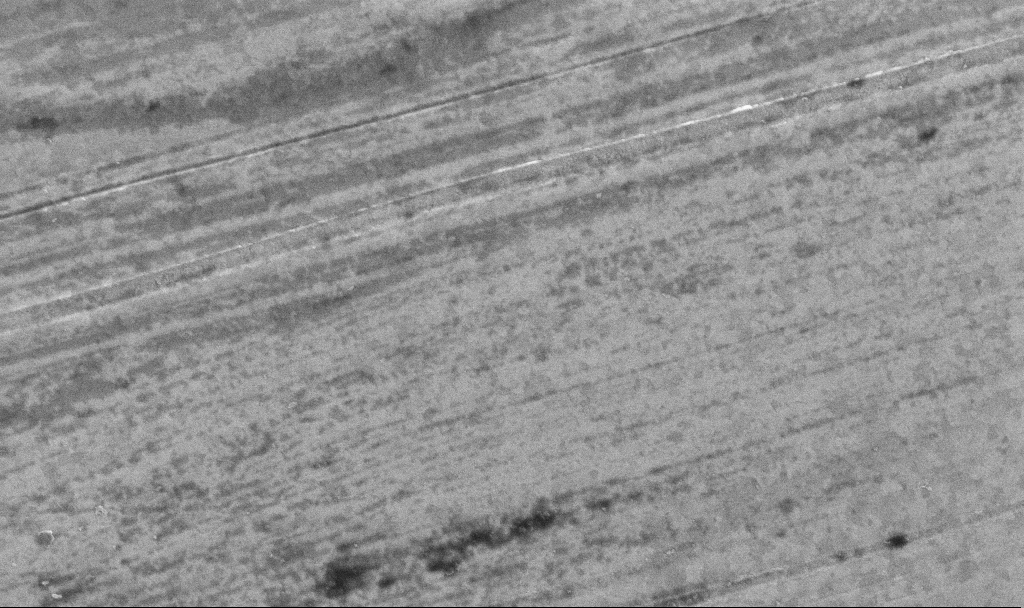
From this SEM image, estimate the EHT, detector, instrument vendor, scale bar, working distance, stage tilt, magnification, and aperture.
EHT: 10 kV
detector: InLens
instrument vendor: Zeiss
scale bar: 1000 nm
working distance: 3.4 mm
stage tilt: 0°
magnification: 50.94 K X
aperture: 30 µm